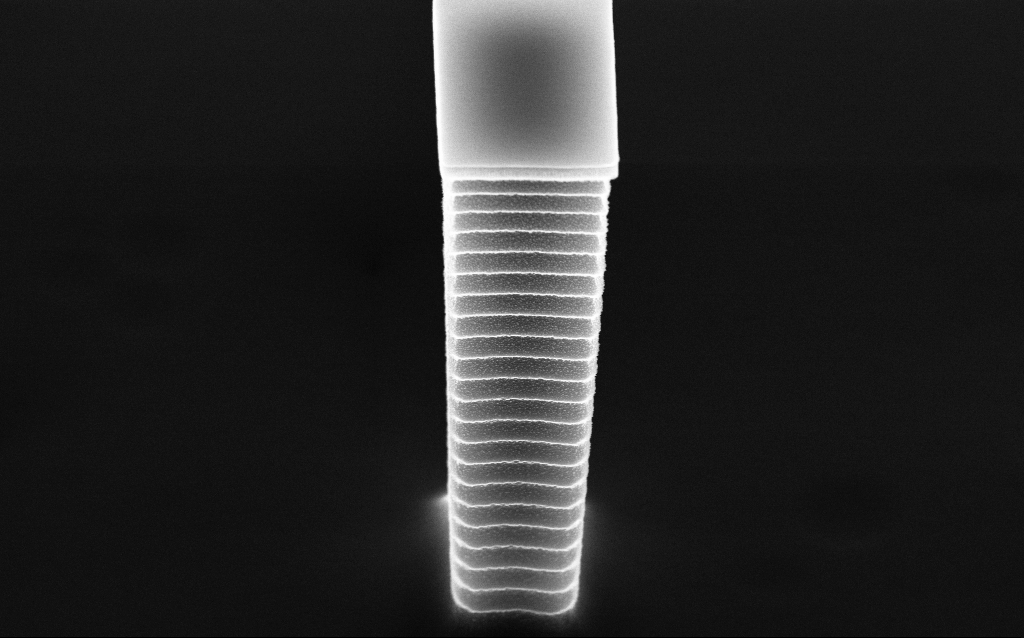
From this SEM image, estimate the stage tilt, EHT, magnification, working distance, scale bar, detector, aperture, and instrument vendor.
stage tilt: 45°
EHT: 10 kV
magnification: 32.46 K X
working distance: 4.8 mm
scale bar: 2000 nm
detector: InLens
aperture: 30 µm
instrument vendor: Zeiss